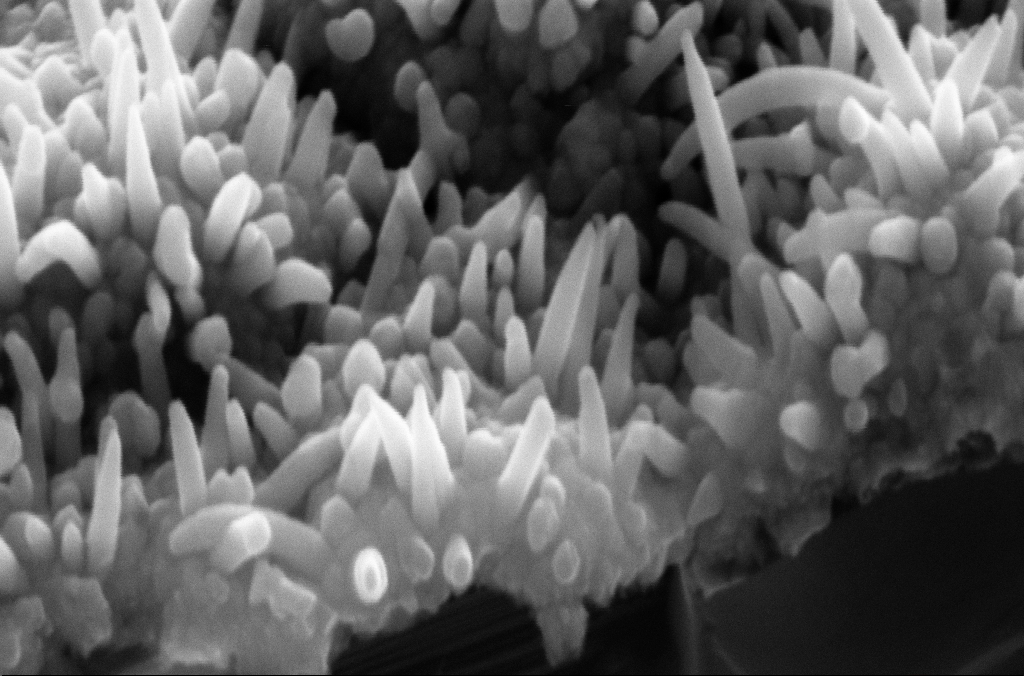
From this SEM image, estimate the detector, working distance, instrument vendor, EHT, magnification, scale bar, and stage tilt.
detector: SE2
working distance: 8 mm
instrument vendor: Zeiss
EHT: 10 kV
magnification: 167.27 K X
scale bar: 200 nm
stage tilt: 45°